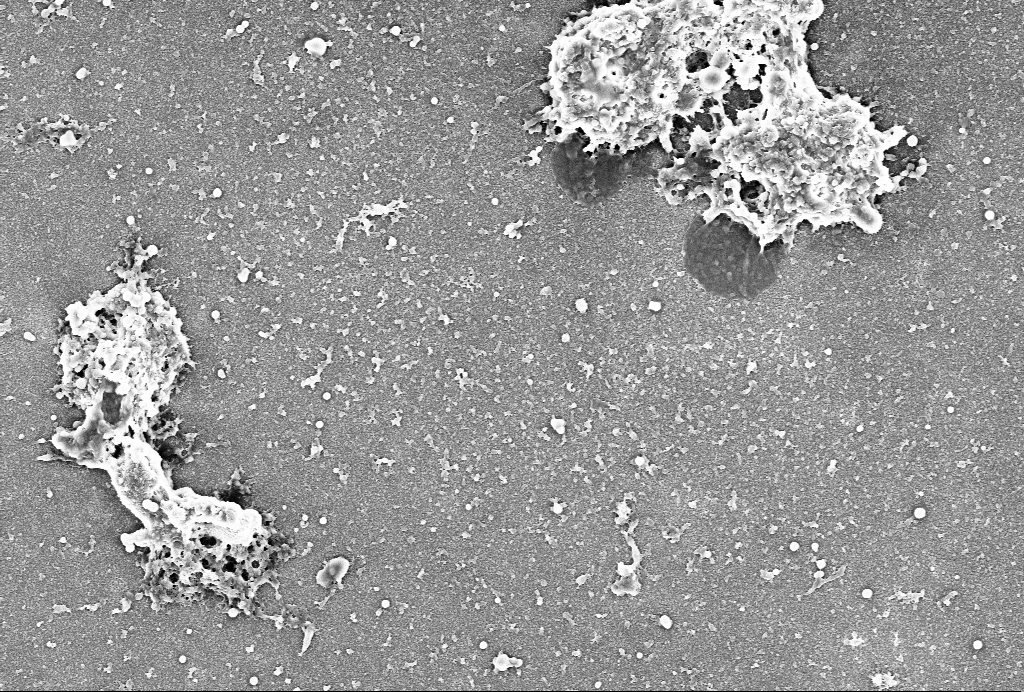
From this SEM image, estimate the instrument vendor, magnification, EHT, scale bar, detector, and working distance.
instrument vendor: Zeiss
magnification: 10 K X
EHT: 4 kV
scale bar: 2000 nm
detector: SE2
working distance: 6 mm